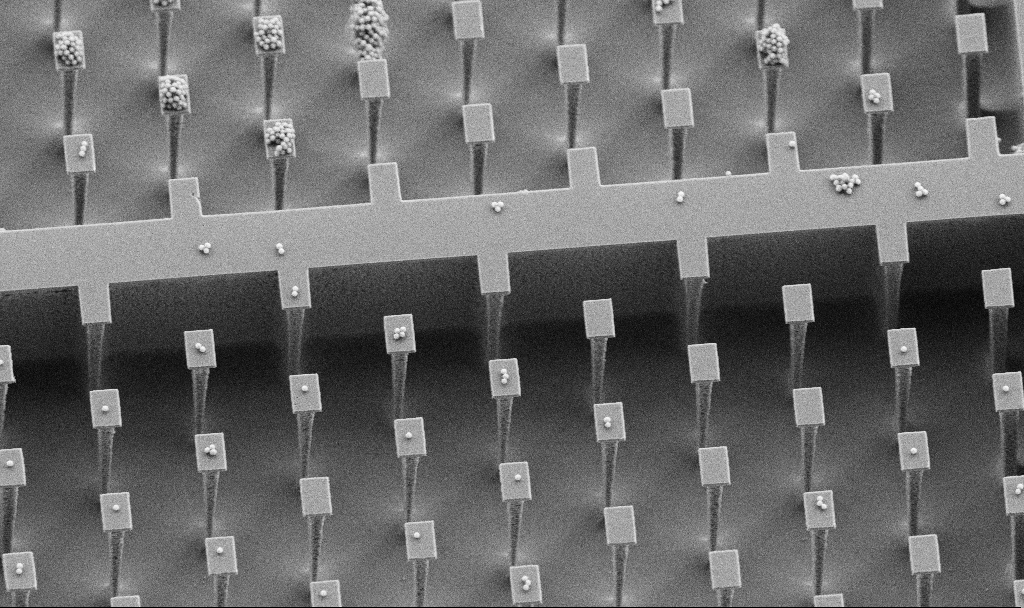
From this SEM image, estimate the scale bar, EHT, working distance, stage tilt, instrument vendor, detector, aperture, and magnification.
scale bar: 10000 nm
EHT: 5 kV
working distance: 4.5 mm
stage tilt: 30°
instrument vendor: Zeiss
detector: SE2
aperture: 30 µm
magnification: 3.66 K X